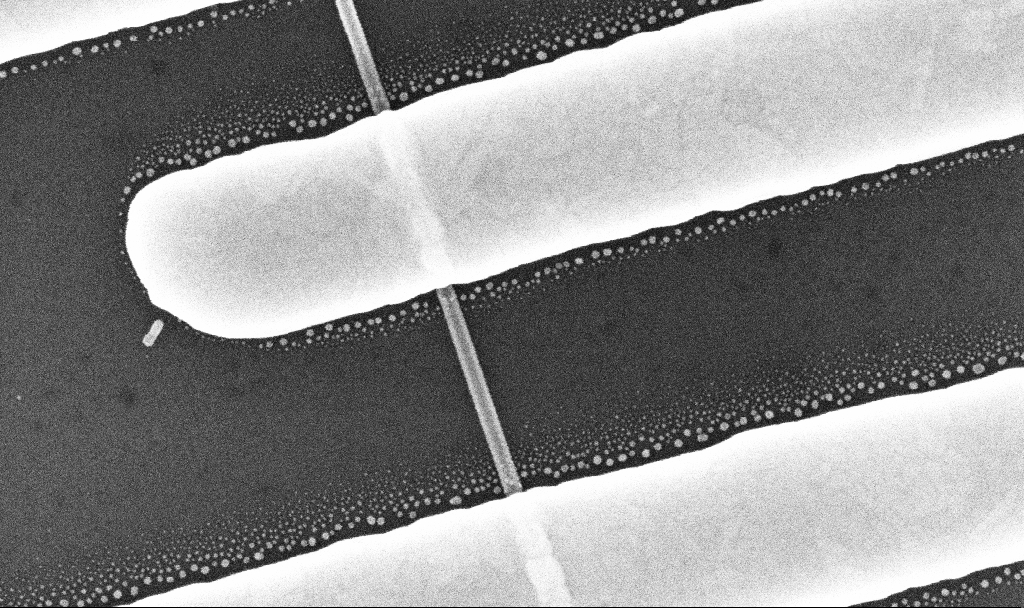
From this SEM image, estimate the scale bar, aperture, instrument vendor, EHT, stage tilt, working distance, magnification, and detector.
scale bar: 200 nm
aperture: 30 µm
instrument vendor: Zeiss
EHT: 10 kV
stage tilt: -0°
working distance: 7 mm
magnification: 168.67 K X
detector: InLens